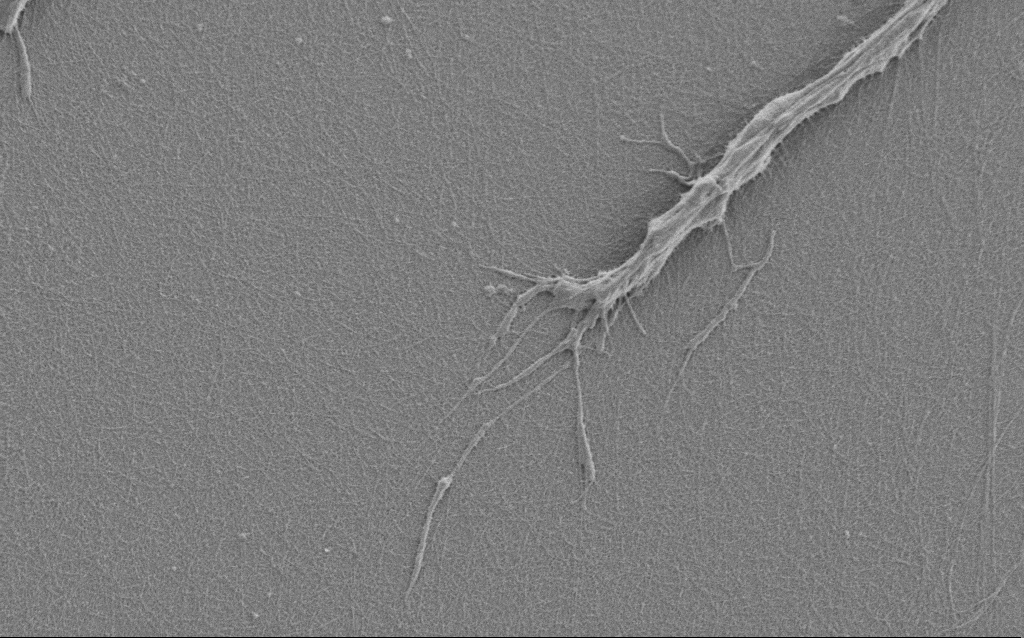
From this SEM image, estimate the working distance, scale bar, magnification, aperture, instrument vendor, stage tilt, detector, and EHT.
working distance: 6 mm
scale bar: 2000 nm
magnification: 7.5 K X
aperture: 30 µm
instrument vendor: Zeiss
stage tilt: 0°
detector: SE2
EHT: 1 kV